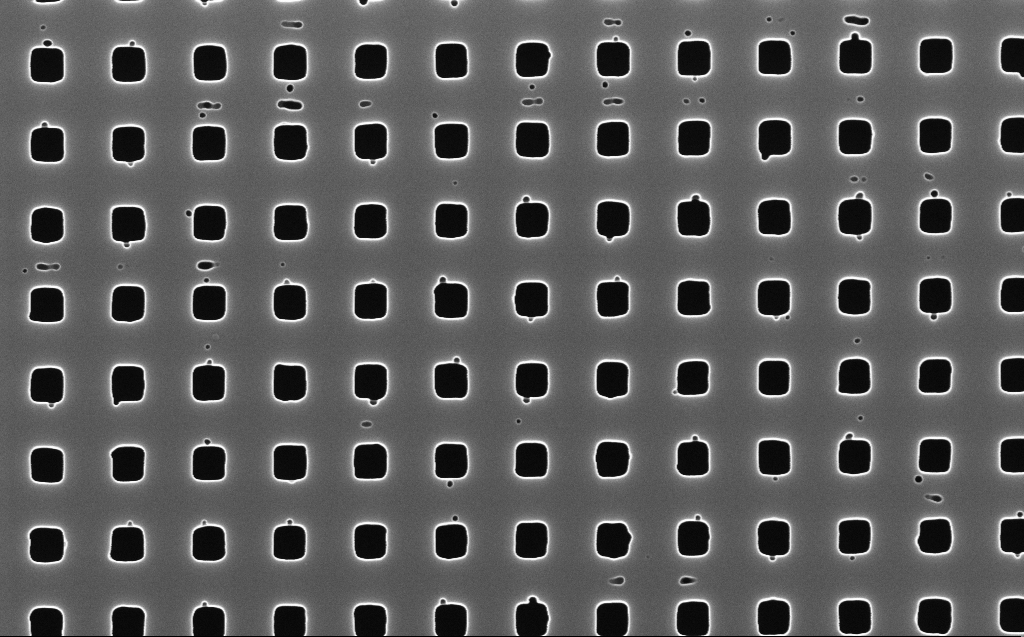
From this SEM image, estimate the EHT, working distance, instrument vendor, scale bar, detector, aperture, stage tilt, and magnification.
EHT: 10 kV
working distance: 5 mm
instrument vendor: Zeiss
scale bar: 1000 nm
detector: InLens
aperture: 30 µm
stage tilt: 0°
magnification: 60 K X